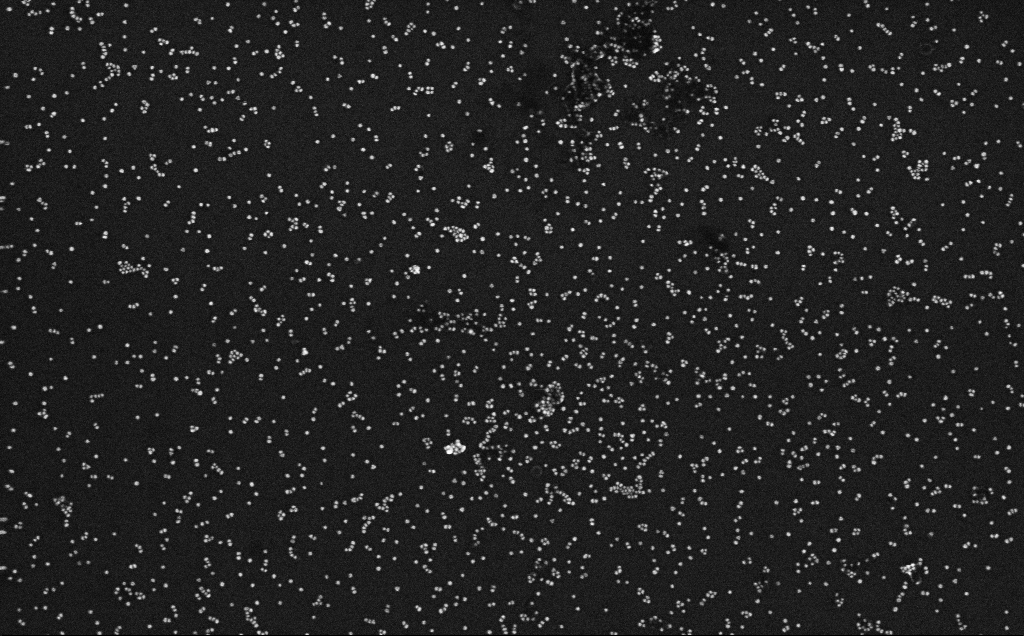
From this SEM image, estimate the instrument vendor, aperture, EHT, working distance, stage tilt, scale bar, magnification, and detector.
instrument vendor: Zeiss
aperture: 30 µm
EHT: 10 kV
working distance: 3.3 mm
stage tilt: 0°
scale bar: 200 nm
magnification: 100 K X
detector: InLens